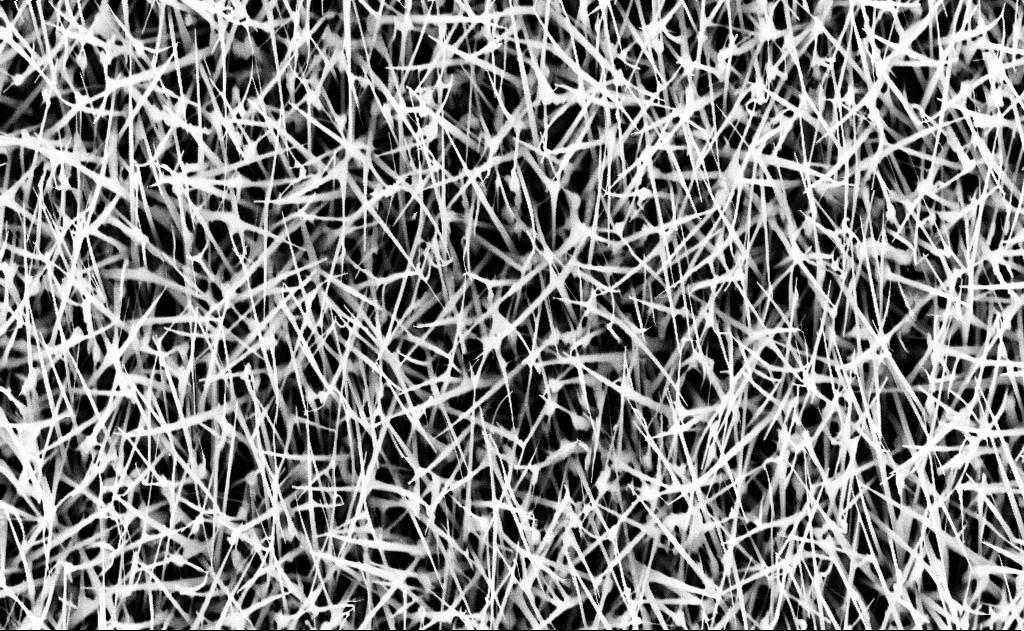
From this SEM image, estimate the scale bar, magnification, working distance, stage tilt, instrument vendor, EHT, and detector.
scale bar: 2000 nm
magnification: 20 K X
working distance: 16 mm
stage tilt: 0°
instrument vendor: Zeiss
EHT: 10 kV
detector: InLens